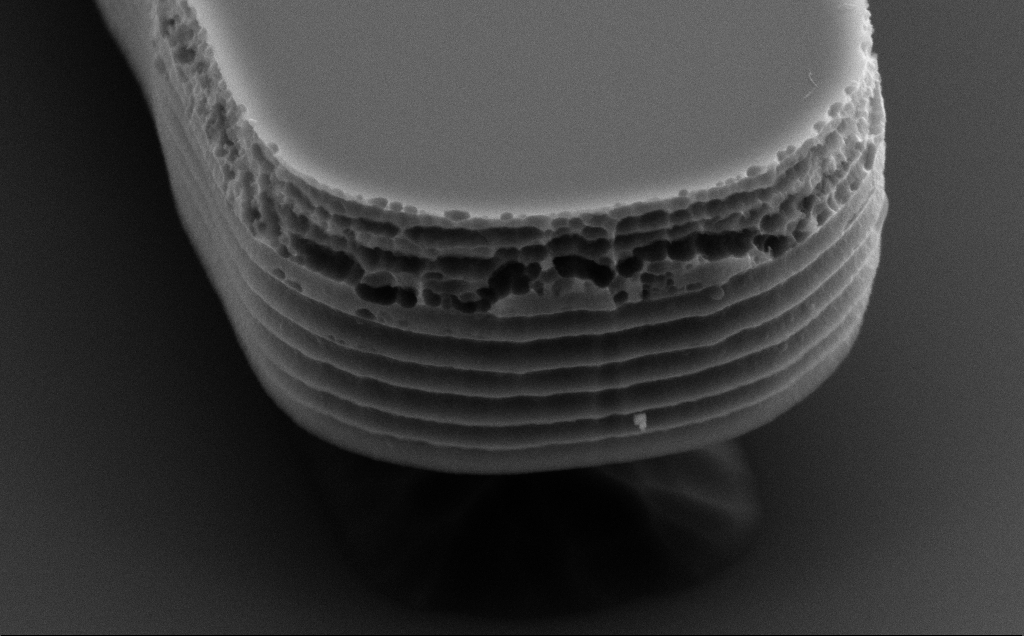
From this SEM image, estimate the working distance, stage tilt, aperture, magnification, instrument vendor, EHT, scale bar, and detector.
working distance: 10 mm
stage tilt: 50°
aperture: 30 µm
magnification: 49.67 K X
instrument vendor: Zeiss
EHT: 5 kV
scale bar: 1000 nm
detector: SE2